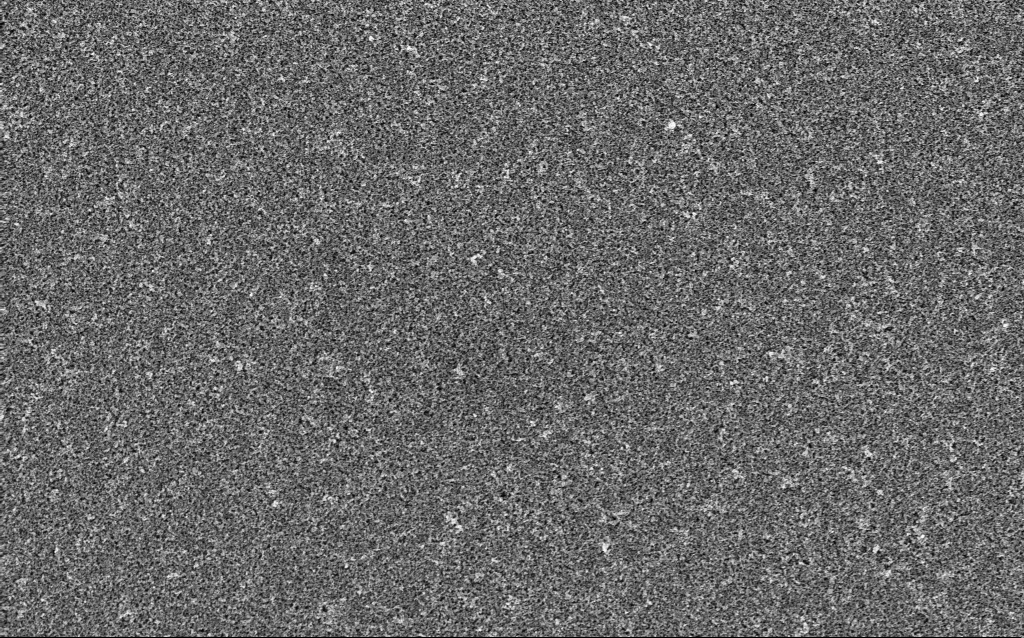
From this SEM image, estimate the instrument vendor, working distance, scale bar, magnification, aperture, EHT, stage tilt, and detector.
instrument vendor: Zeiss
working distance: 4.9 mm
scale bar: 1000 nm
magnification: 15.33 K X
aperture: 30 µm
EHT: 5 kV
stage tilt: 0°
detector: InLens